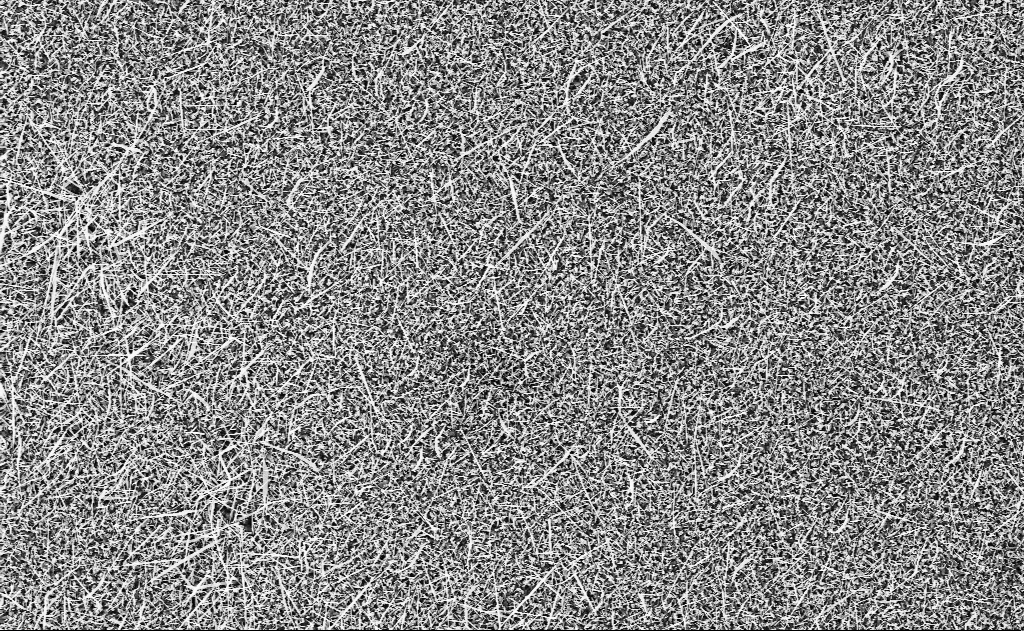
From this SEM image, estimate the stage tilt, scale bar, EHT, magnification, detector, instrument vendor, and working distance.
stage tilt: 0°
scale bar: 10000 nm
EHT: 10 kV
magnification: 5 K X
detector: InLens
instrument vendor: Zeiss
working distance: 15 mm